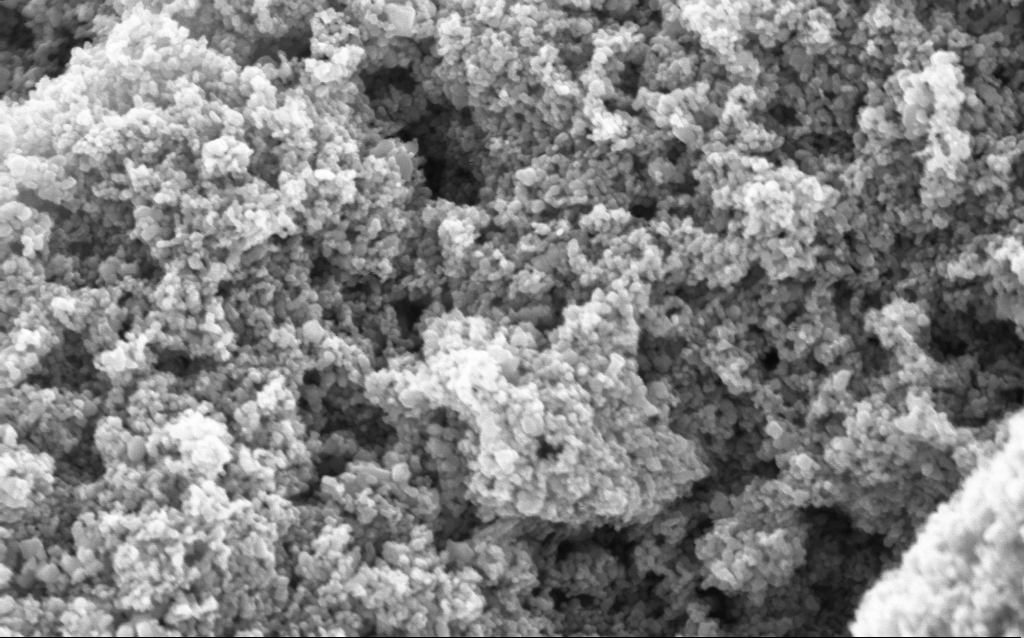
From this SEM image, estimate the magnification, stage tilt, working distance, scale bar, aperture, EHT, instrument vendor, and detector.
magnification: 114.65 K X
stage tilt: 0°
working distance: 4.7 mm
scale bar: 200 nm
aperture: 30 µm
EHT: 5 kV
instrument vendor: Zeiss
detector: InLens